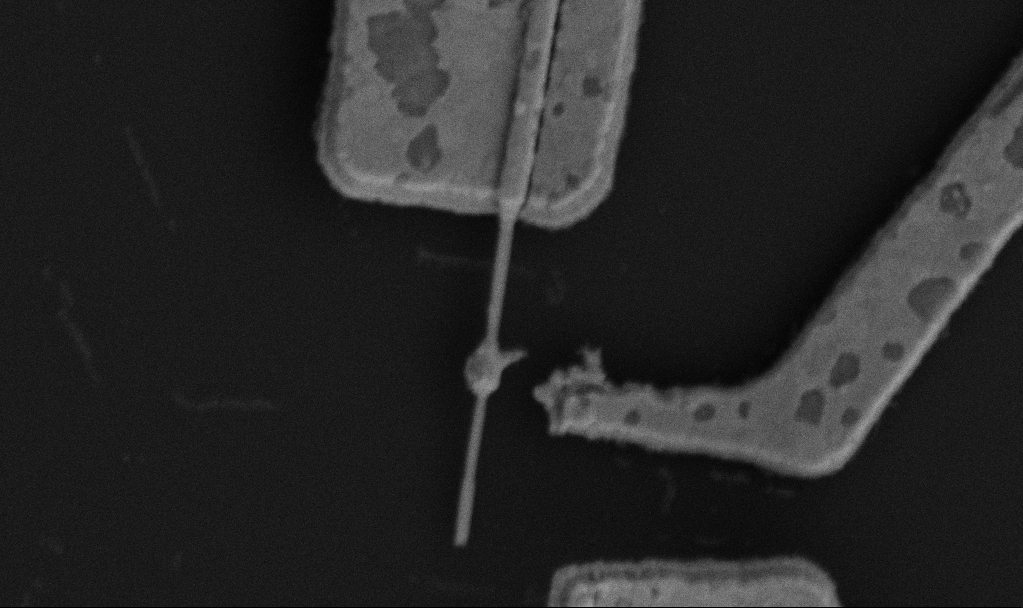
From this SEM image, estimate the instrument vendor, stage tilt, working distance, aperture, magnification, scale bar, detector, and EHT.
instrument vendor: Zeiss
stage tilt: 0°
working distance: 10.4 mm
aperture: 30 µm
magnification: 50 K X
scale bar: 1000 nm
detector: SE2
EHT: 5 kV